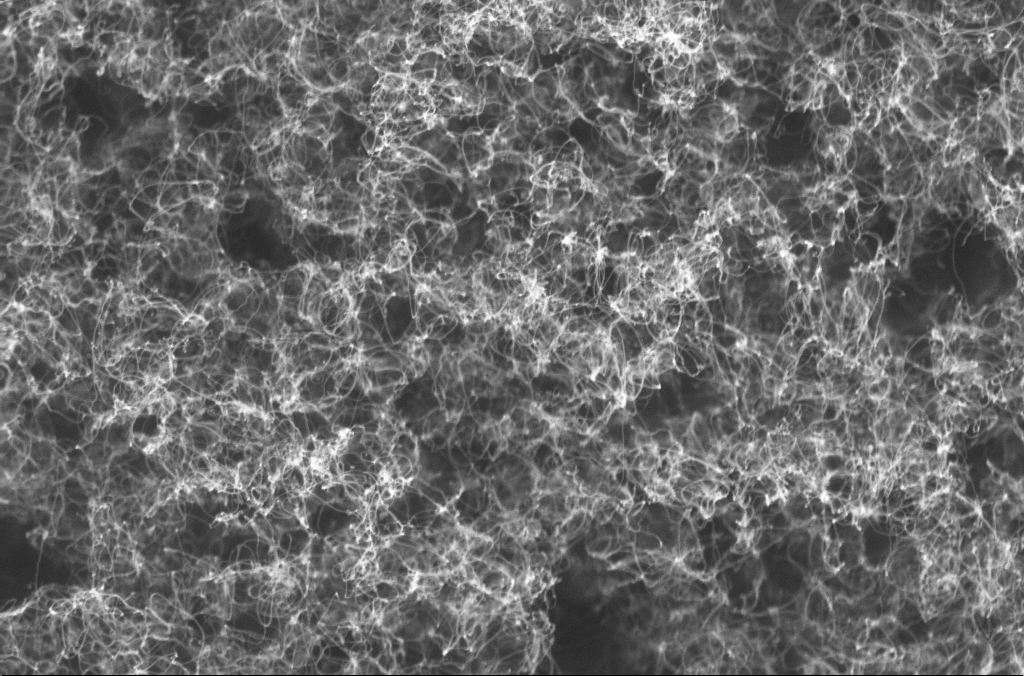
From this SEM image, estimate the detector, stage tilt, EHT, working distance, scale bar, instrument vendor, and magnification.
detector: InLens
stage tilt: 0°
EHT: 10 kV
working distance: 3.9 mm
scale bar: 1000 nm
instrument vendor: Zeiss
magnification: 50 K X